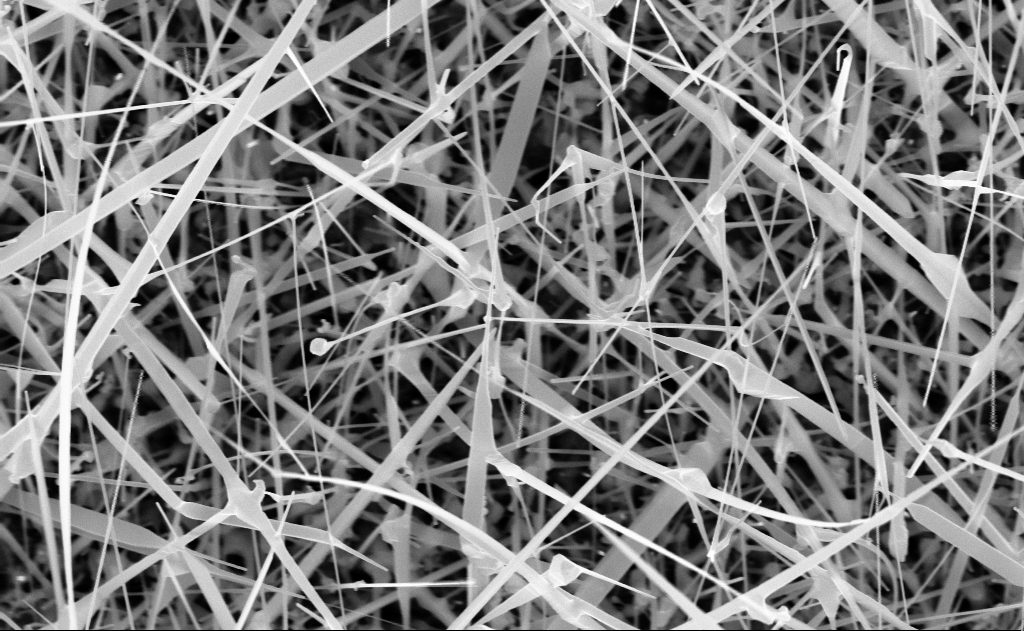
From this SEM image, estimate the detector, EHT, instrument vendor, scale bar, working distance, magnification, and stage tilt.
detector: InLens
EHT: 10 kV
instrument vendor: Zeiss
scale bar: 1000 nm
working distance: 10 mm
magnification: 40 K X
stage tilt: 0°